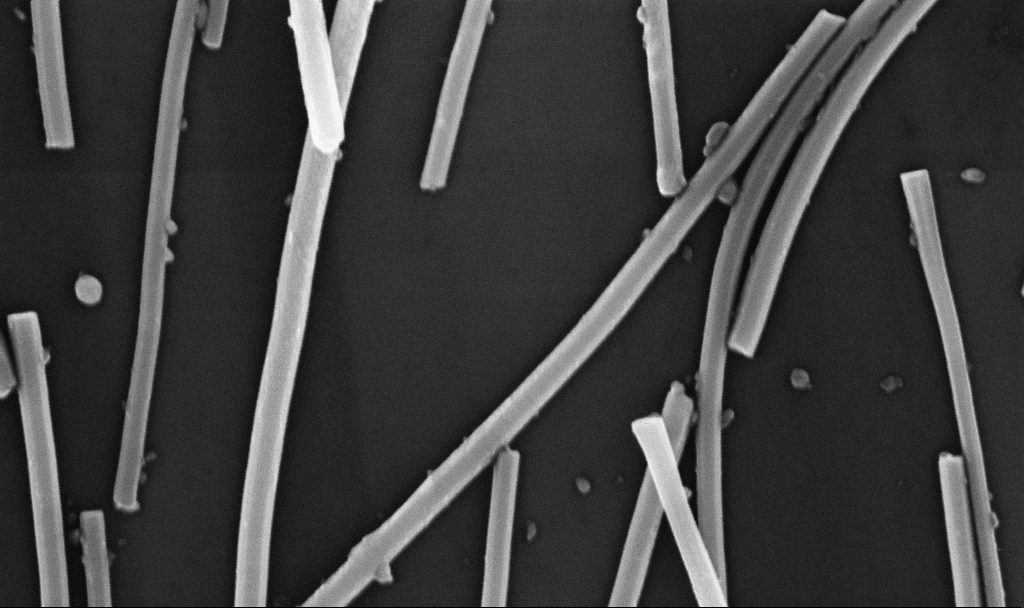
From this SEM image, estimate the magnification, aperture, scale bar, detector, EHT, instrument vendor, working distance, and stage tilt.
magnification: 94.6 K X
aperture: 30 µm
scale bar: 200 nm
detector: InLens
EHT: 10 kV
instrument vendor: Zeiss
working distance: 6.7 mm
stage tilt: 0°